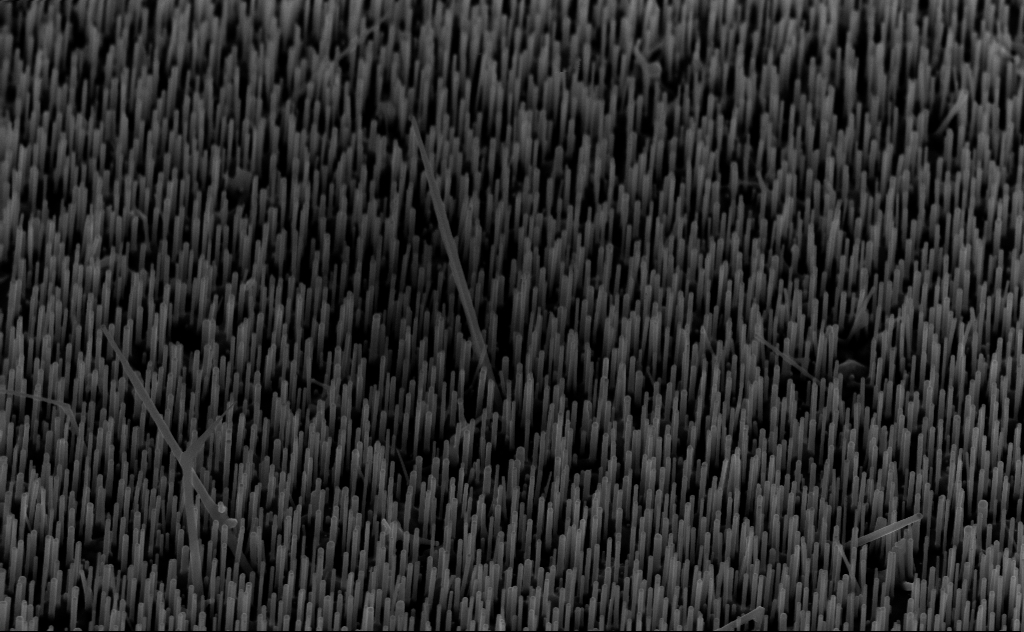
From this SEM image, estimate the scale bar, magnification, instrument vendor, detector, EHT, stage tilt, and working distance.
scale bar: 1000 nm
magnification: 40 K X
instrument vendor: Zeiss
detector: InLens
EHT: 10 kV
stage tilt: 45°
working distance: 6 mm